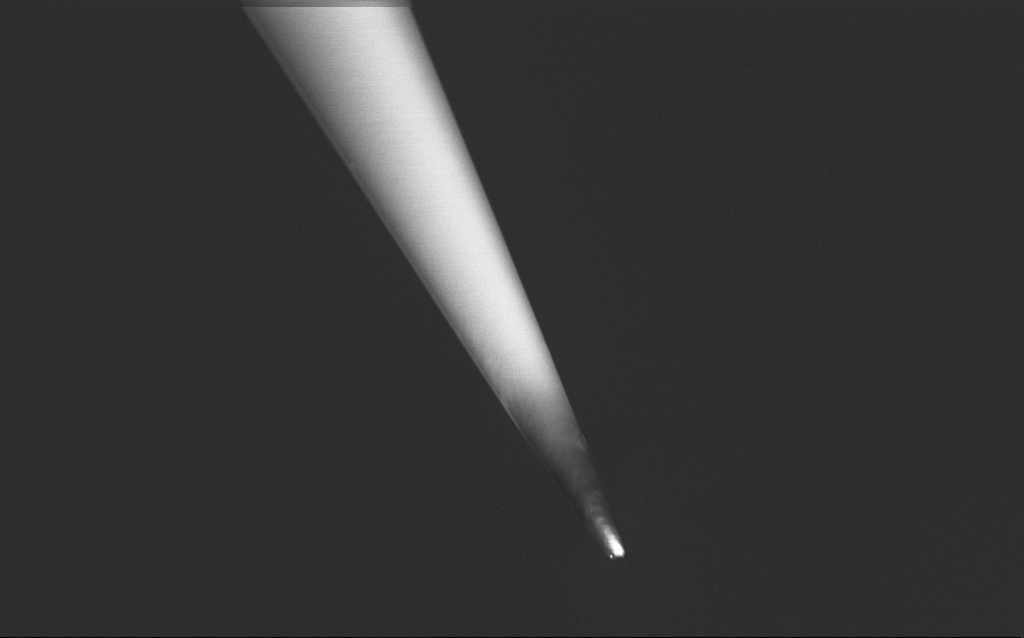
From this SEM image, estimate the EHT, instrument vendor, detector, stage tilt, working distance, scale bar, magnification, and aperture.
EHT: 1 kV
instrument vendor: Zeiss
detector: InLens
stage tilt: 45°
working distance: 6 mm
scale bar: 2000 nm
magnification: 10 K X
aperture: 30 µm